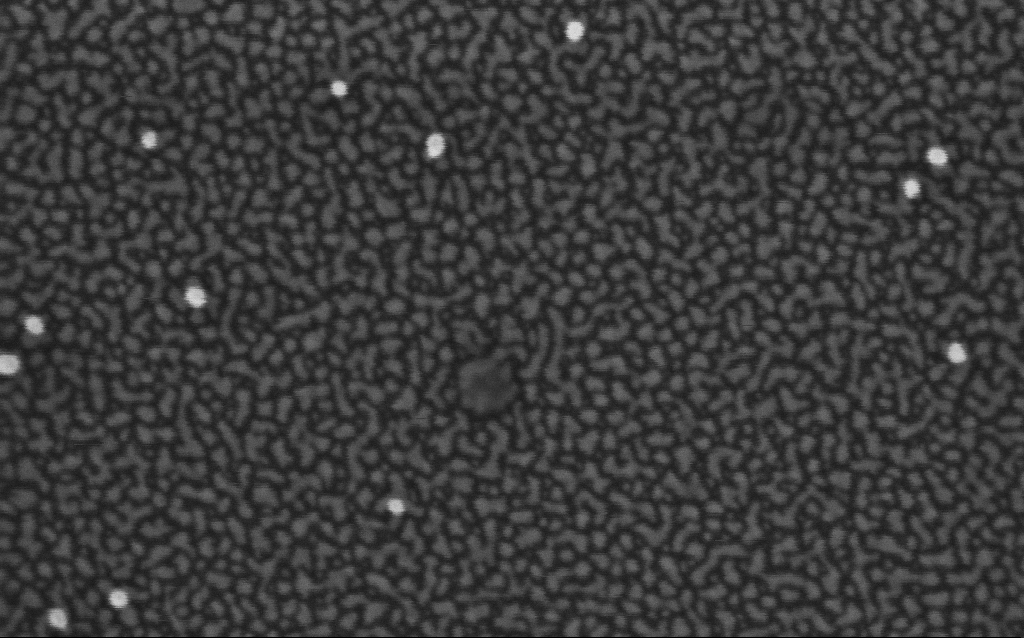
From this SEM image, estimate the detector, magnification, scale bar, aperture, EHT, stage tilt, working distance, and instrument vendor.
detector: InLens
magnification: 400 K X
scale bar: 100 nm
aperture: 30 µm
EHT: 1 kV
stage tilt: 0°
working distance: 1.7 mm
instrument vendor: Zeiss